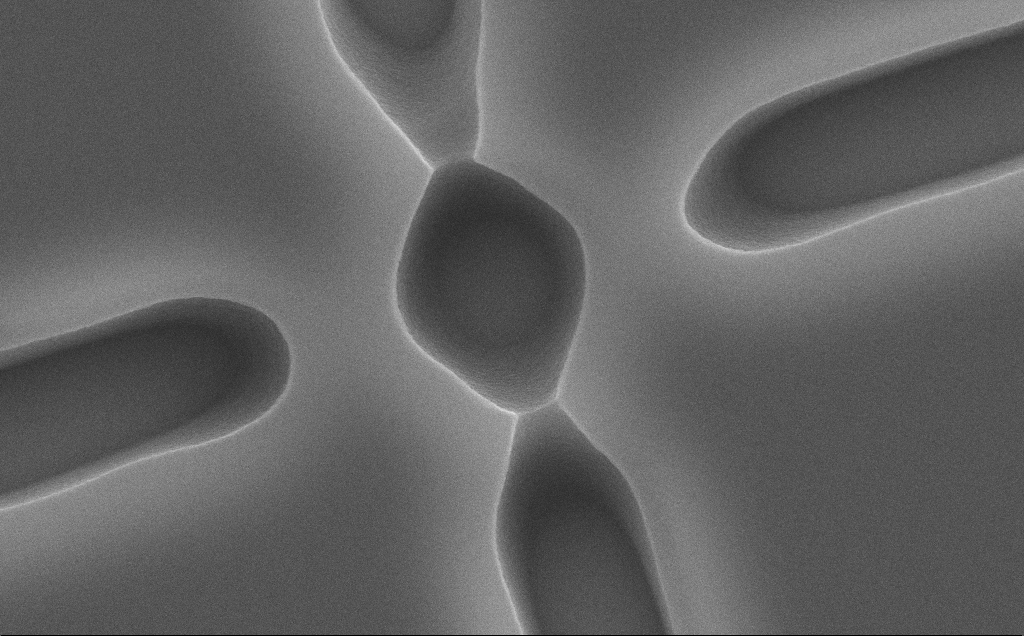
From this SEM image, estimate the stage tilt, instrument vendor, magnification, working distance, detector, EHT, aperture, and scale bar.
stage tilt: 0°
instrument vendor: Zeiss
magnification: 27.64 K X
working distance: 12 mm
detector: SE2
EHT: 10 kV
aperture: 30 µm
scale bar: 2000 nm